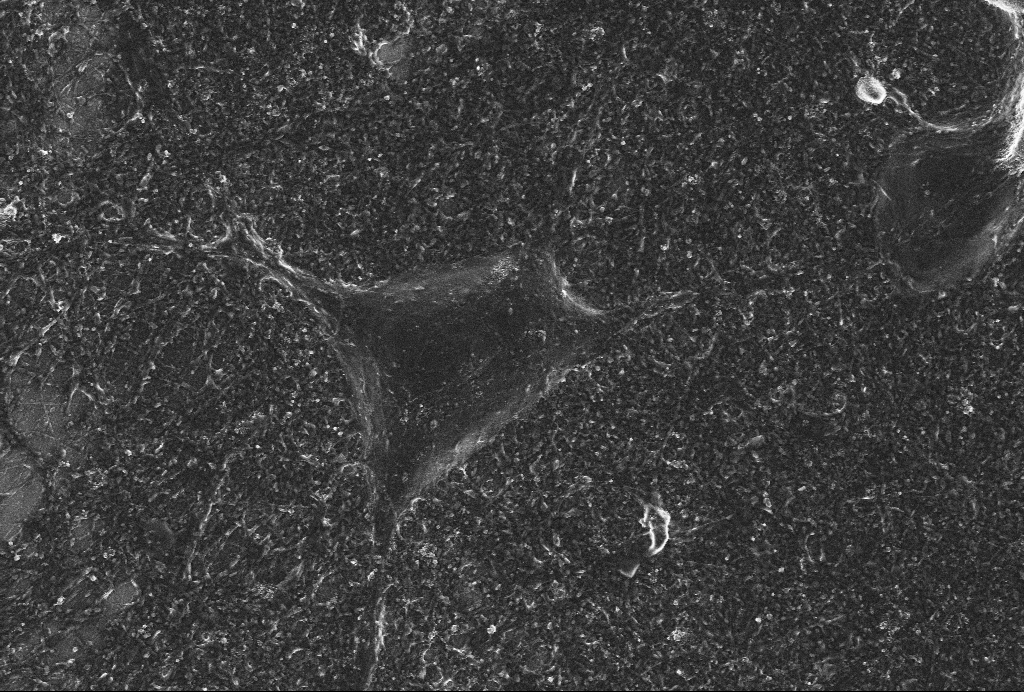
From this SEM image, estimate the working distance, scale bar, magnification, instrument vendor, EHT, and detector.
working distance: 6 mm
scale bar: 10000 nm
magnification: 4 K X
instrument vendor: Zeiss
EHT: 4 kV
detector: SE2